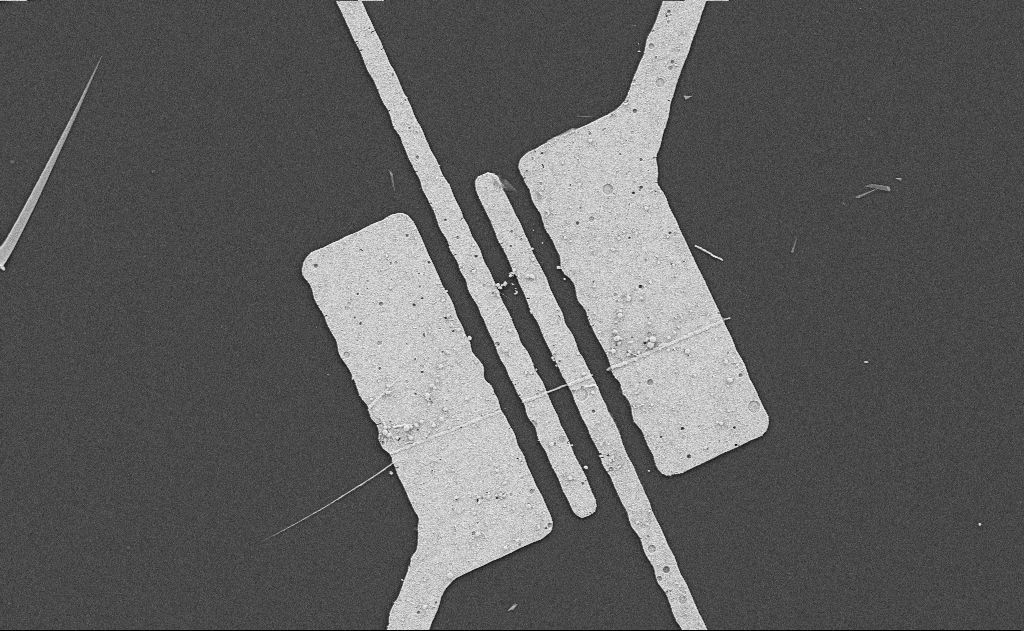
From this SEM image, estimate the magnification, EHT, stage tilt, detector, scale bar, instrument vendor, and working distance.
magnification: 4.41 K X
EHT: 5 kV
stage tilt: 0°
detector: SE2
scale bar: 10000 nm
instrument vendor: Zeiss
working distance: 7 mm